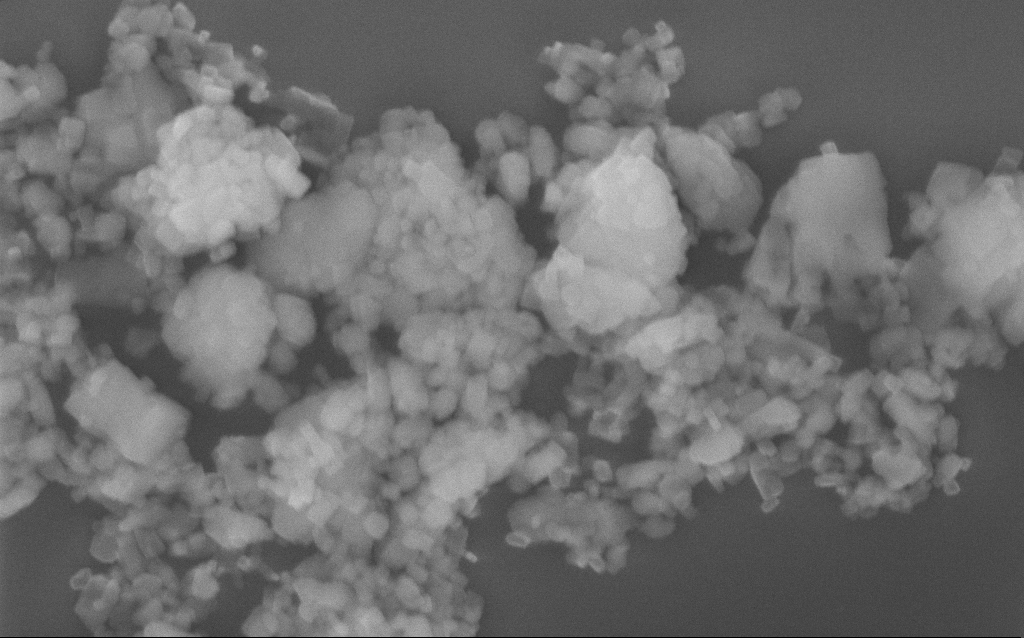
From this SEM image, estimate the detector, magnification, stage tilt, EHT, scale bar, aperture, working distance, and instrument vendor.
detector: InLens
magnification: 77.09 K X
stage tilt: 0°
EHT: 10 kV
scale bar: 200 nm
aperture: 30 µm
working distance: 3 mm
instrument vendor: Zeiss